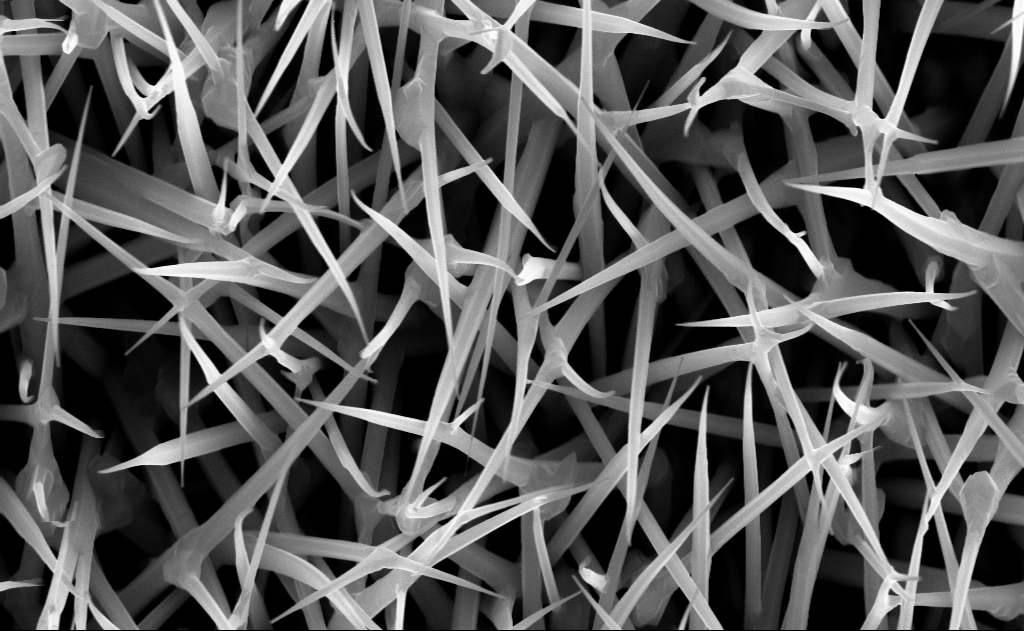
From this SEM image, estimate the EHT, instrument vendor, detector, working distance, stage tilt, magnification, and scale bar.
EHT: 10 kV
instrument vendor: Zeiss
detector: InLens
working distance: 7 mm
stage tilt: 0°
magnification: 40 K X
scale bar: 1000 nm